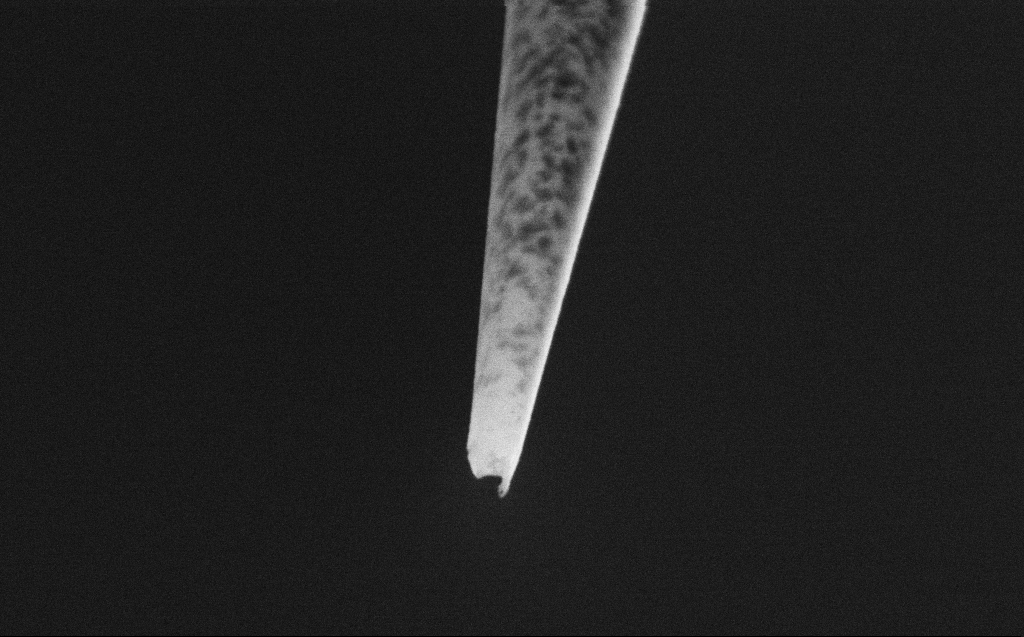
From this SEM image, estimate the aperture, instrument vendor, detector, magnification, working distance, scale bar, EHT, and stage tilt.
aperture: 30 µm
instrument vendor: Zeiss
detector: SE2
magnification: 100 K X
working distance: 4 mm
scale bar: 200 nm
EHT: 2 kV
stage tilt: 45°